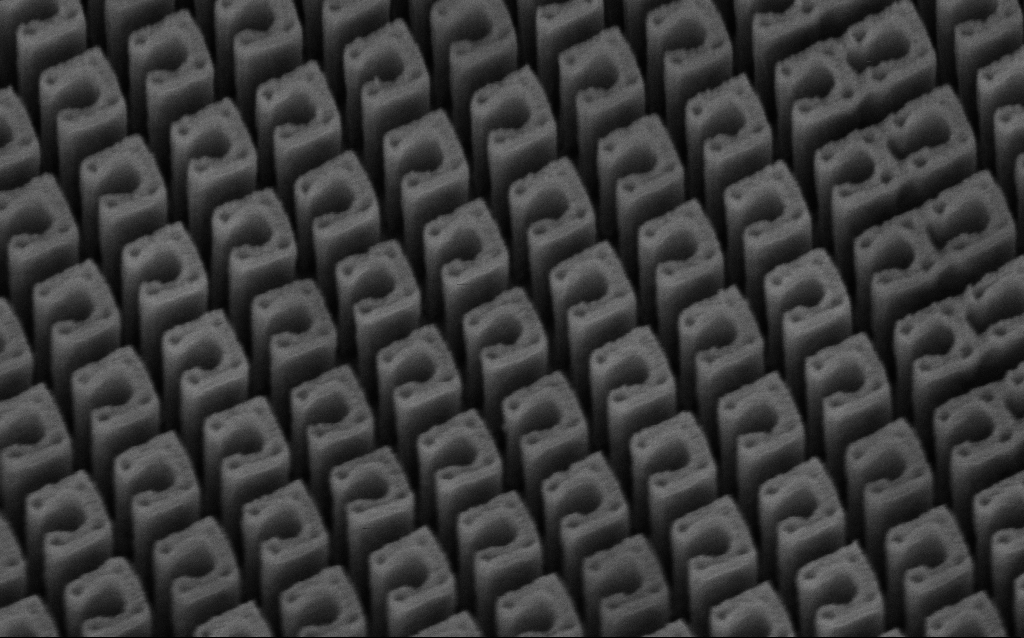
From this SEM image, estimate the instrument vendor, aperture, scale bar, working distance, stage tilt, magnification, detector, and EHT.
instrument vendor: Zeiss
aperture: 30 µm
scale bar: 200 nm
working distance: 7.3 mm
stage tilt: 45°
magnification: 76.65 K X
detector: InLens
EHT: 3 kV